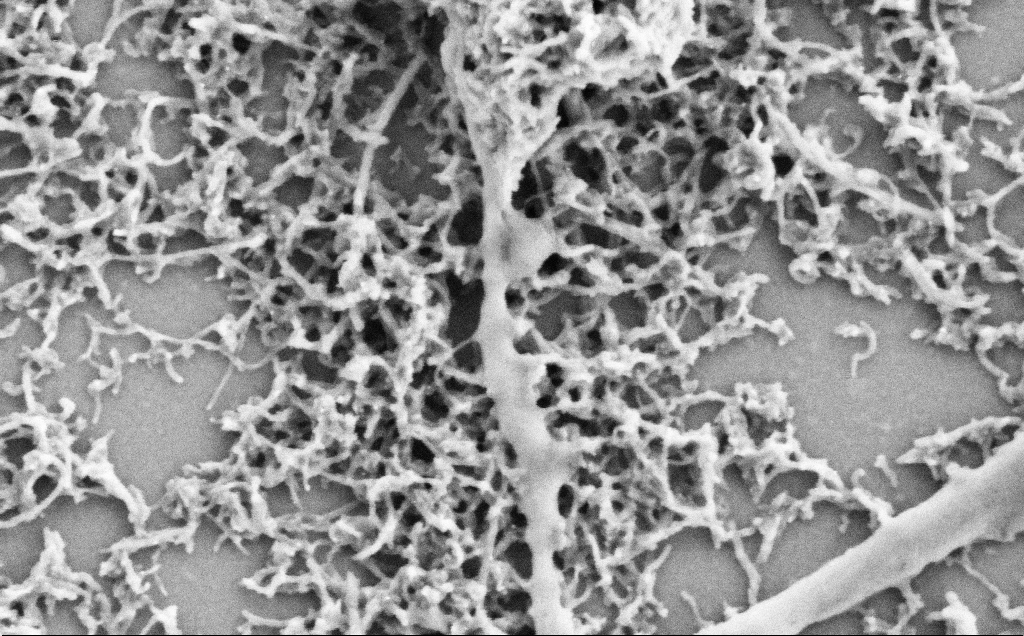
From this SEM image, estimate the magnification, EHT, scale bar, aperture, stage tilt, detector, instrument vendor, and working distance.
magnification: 75 K X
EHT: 2 kV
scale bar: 200 nm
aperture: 30 µm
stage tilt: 0°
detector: SE2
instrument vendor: Zeiss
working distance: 7.1 mm